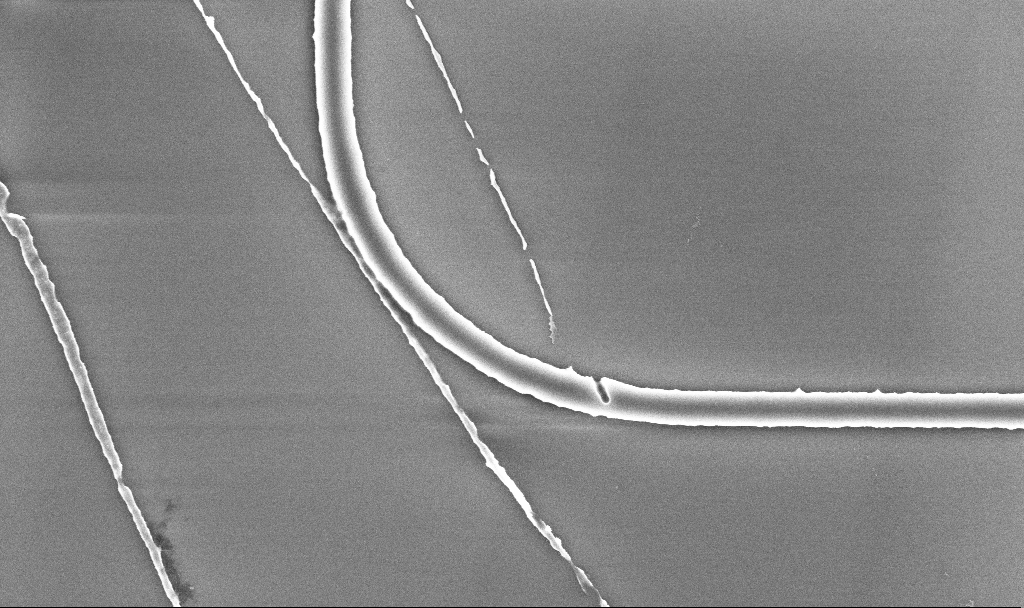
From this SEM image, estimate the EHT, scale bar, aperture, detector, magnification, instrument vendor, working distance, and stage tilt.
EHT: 5 kV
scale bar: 2000 nm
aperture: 30 µm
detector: InLens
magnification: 26.62 K X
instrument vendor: Zeiss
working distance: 5.2 mm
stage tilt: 0°